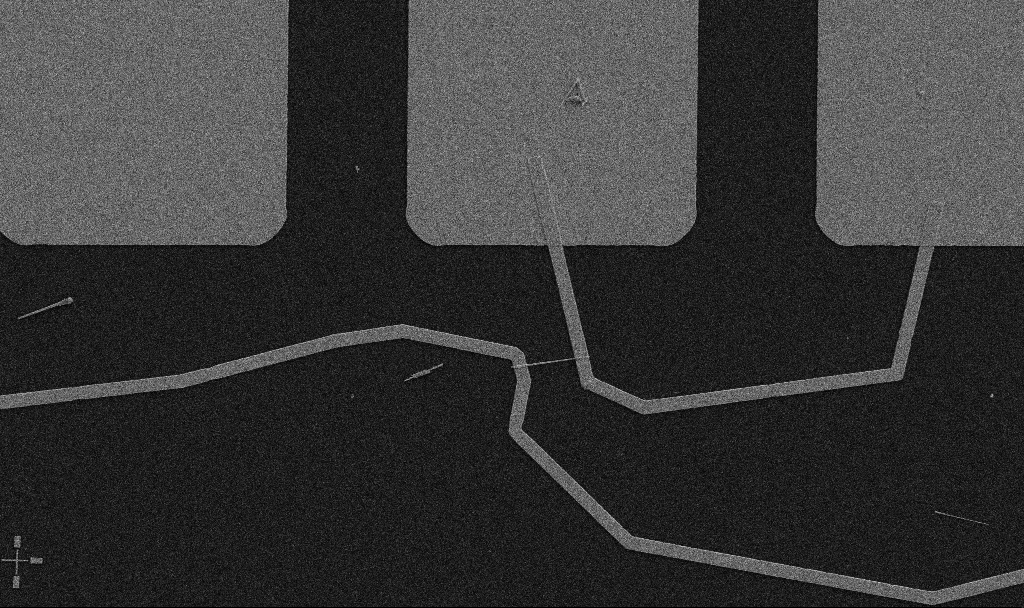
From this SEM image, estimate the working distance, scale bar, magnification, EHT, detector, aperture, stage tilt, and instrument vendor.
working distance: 10.7 mm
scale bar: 10000 nm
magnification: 5 K X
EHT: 5 kV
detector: SE2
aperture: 30 µm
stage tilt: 0°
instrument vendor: Zeiss